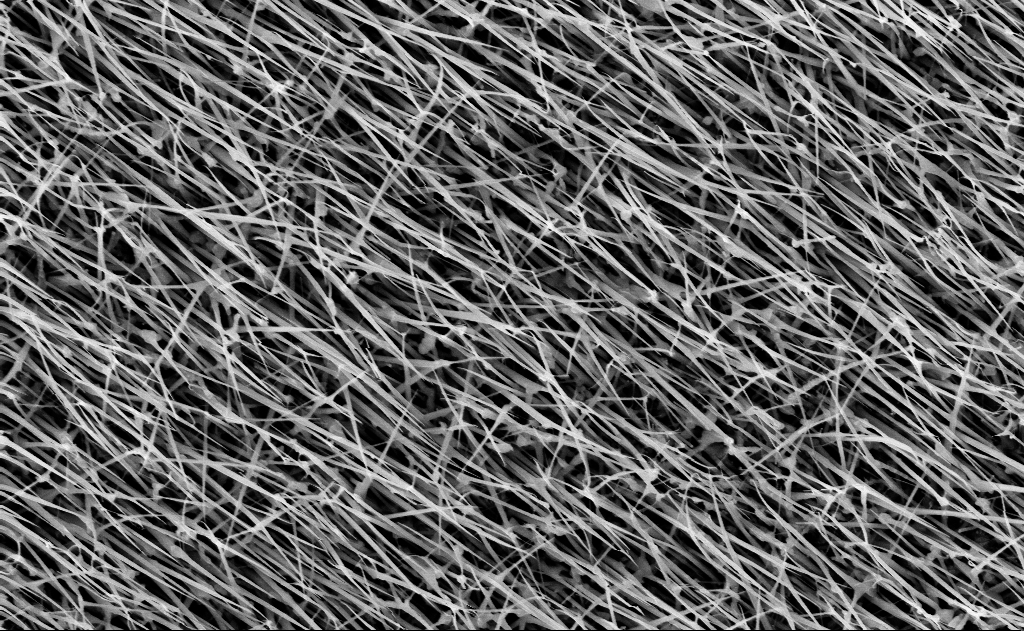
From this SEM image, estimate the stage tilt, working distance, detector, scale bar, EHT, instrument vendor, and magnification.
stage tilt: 0°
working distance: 13 mm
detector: InLens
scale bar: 1000 nm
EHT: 10 kV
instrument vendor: Zeiss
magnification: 20 K X